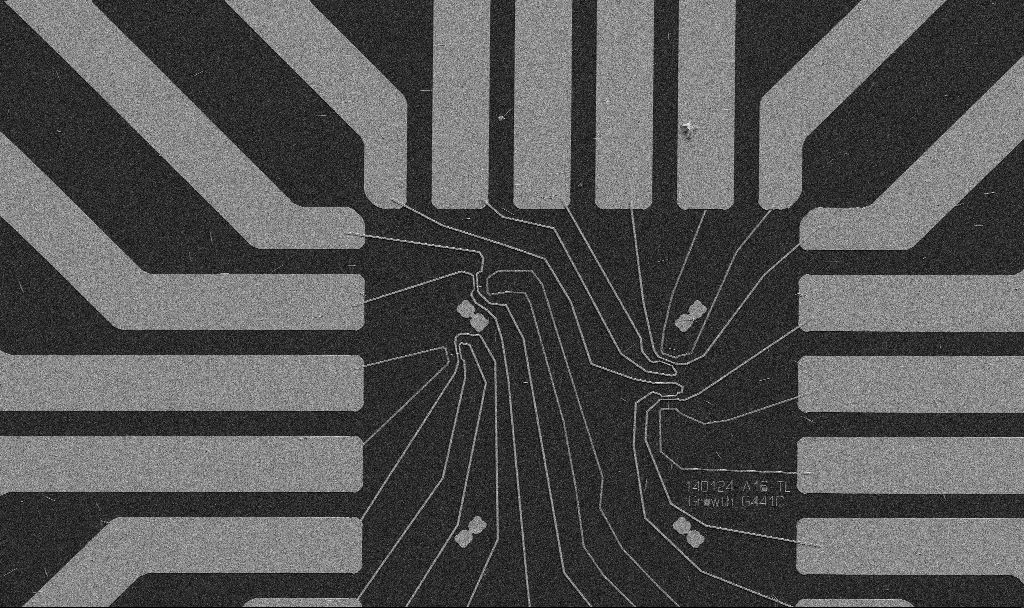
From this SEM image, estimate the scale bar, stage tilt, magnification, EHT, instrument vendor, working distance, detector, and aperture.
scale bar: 20000 nm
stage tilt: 0°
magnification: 1 K X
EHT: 5 kV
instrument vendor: Zeiss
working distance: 10.7 mm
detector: SE2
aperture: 30 µm